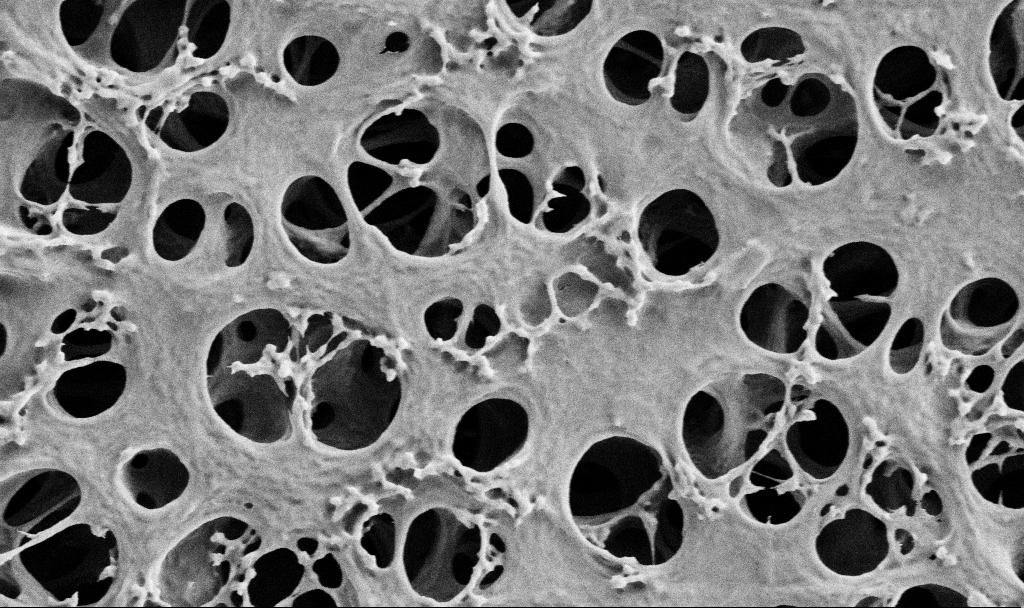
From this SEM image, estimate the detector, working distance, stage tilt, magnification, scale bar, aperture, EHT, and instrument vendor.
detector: SE2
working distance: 3.5 mm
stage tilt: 0°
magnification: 25 K X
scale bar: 2000 nm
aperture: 30 µm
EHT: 2 kV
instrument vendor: Zeiss